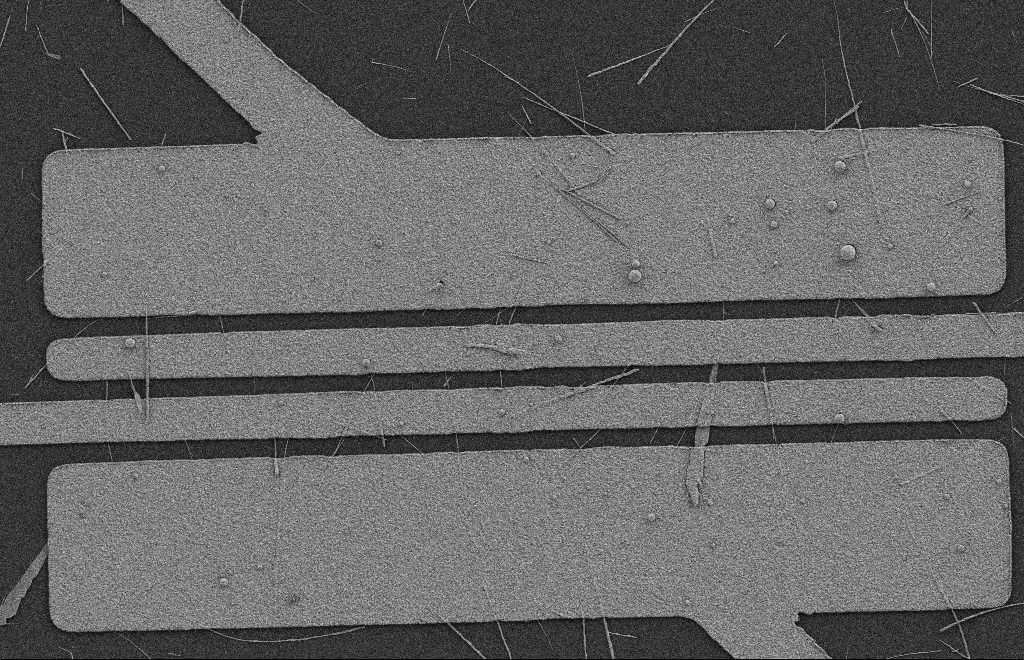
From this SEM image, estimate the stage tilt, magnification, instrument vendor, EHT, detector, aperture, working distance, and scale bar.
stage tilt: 0°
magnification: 5.75 K X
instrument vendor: Zeiss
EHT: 2 kV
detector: SE2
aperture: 20 µm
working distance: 8 mm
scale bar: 2000 nm